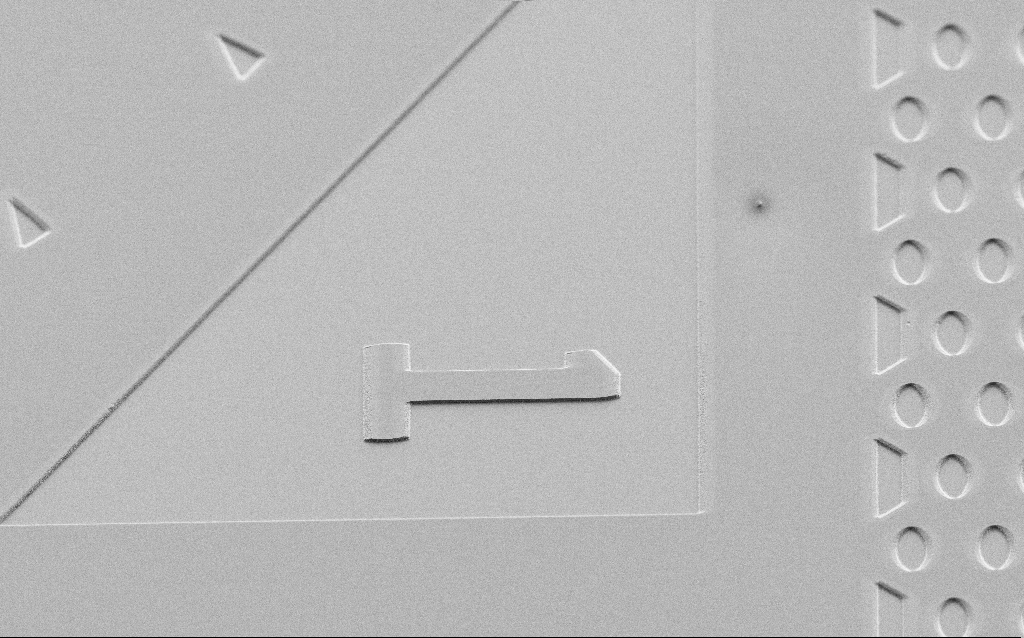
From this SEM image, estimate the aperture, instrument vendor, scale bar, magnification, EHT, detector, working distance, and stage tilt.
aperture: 30 µm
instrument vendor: Zeiss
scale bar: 20000 nm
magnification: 0.884 K X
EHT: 3 kV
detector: SE2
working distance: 6 mm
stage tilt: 45°